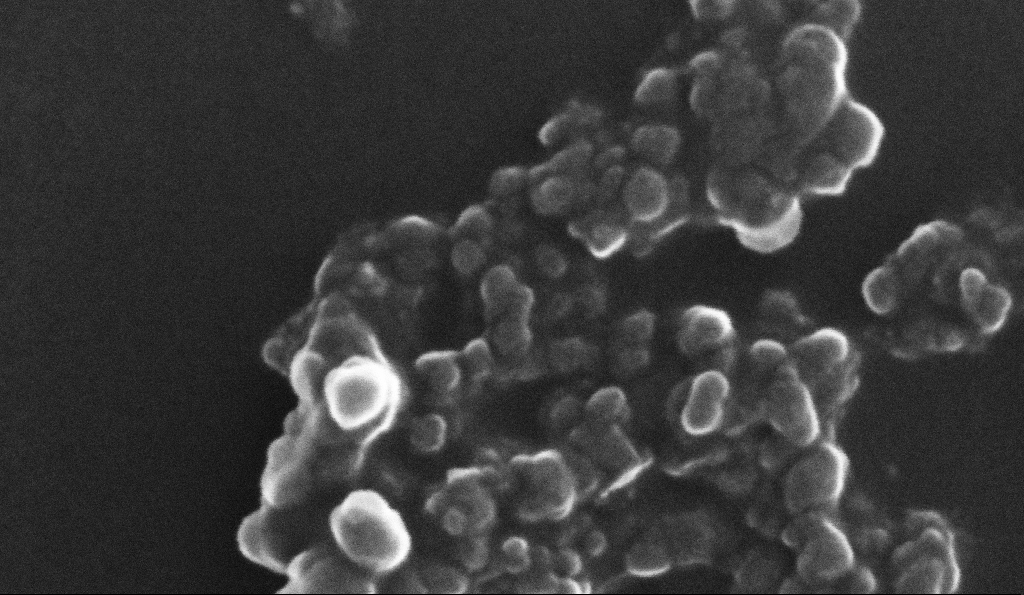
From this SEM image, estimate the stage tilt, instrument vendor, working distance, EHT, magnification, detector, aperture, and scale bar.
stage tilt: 0°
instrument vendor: Zeiss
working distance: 5.3 mm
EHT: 10 kV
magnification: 454.55 K X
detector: InLens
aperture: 30 µm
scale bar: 100 nm